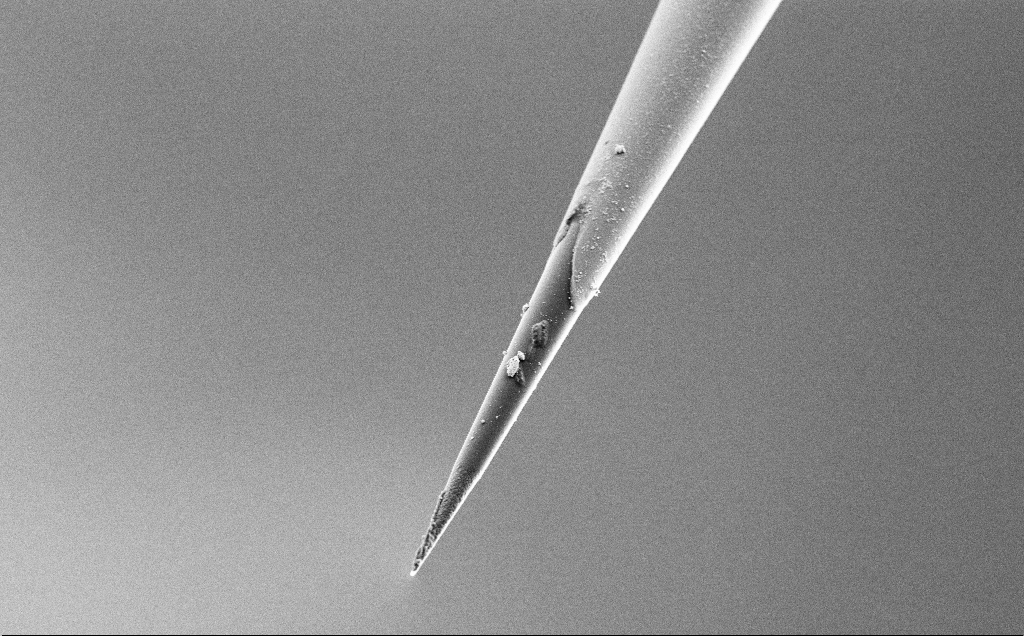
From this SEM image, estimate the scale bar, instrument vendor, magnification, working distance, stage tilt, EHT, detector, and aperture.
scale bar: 10000 nm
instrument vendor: Zeiss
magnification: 5 K X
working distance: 7.7 mm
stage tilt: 45°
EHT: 3 kV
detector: SE2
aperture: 30 µm